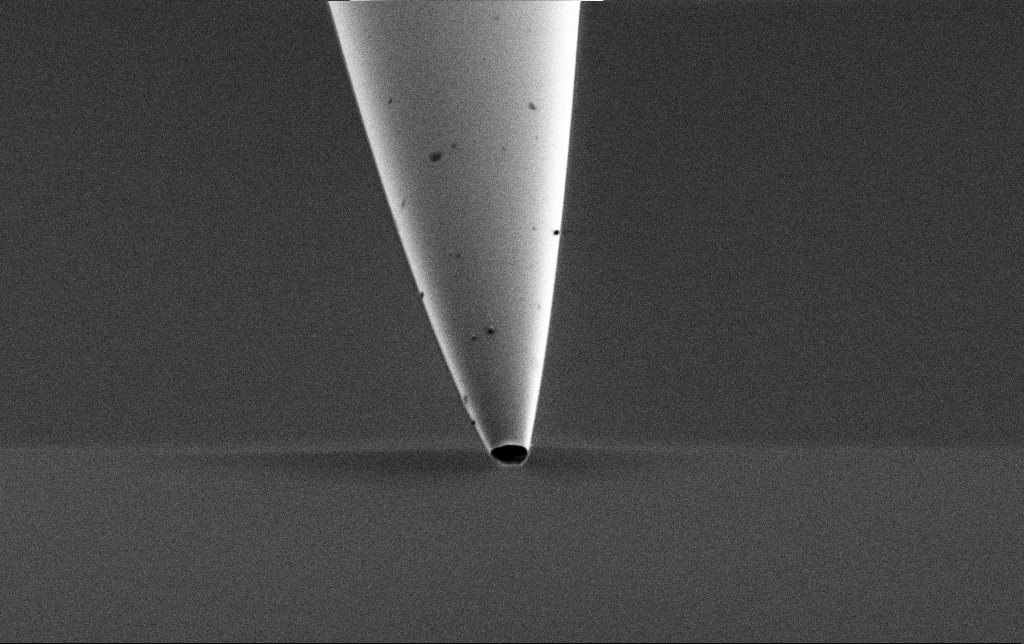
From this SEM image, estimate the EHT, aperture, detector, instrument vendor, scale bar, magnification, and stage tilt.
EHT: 1 kV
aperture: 30 µm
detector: SE2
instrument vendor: Zeiss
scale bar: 2000 nm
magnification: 10 K X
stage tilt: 45°